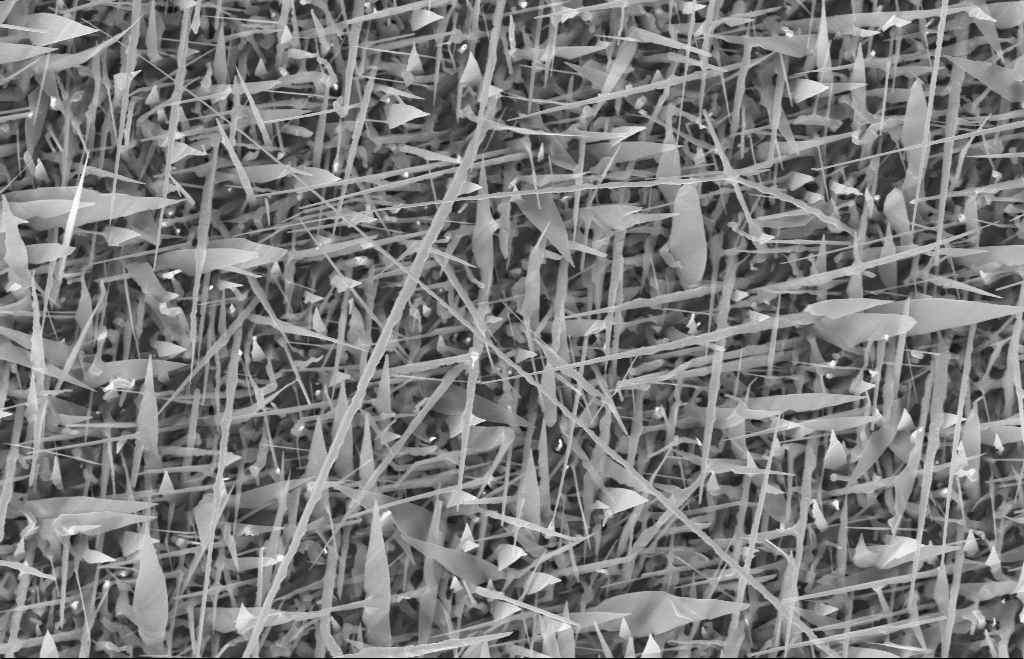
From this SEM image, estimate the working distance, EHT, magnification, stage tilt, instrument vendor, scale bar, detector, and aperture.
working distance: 10 mm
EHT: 10 kV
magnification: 20 K X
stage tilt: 0°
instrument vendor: Zeiss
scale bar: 1000 nm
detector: InLens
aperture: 30 µm